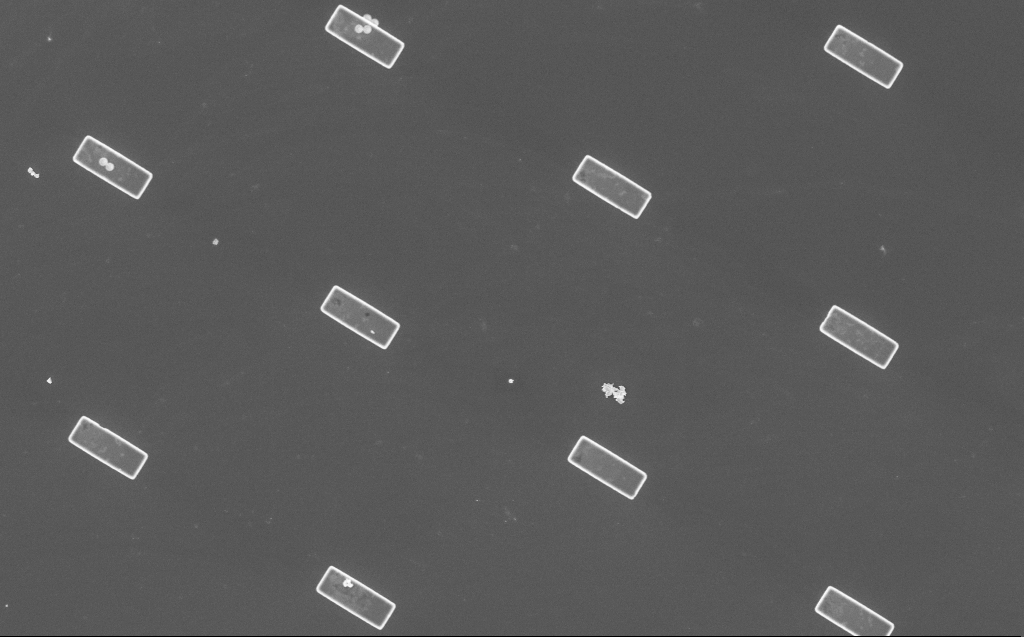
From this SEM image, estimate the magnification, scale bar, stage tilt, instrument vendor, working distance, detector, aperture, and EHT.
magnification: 3.74 K X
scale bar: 10000 nm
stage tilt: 0°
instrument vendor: Zeiss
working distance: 8 mm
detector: InLens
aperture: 30 µm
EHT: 5 kV